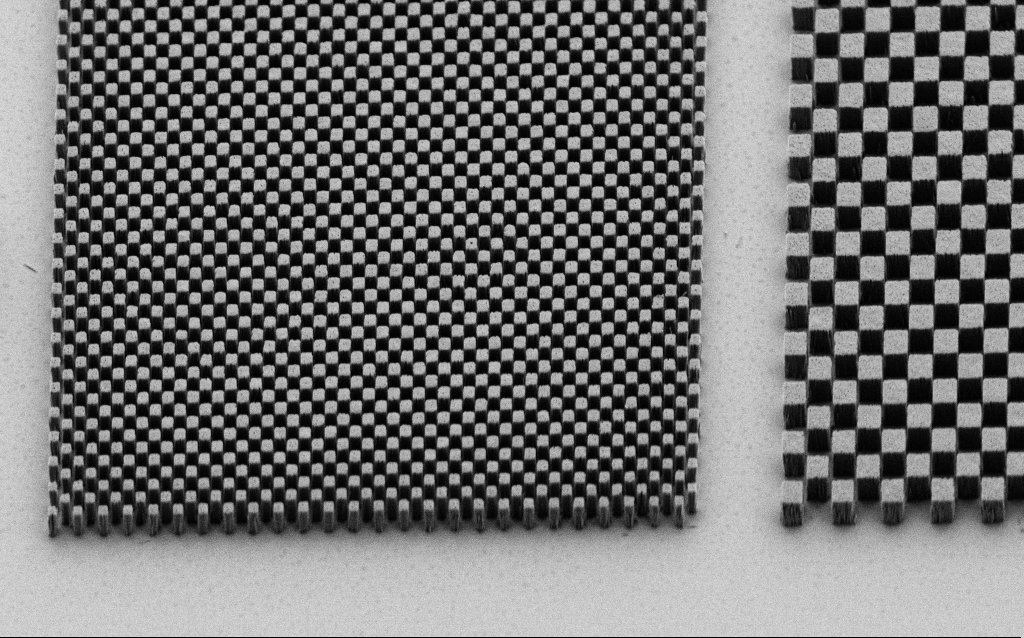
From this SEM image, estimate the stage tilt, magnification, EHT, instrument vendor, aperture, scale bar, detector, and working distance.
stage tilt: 45°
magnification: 9.12 K X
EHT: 1.5 kV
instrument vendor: Zeiss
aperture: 30 µm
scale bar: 2000 nm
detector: SE2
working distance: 7 mm